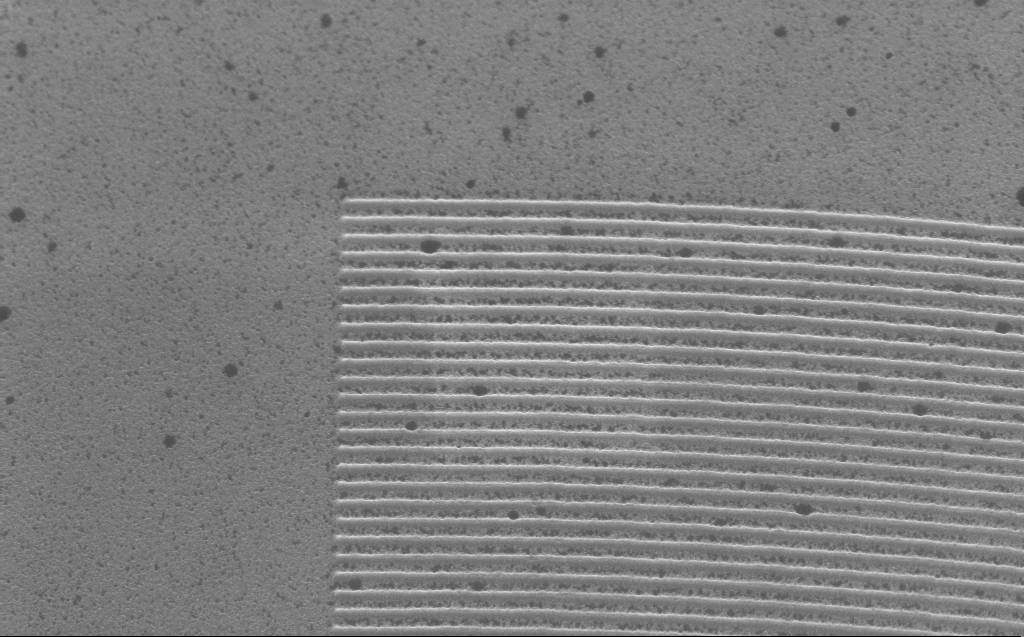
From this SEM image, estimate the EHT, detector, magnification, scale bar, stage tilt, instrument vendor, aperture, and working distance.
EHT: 5 kV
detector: InLens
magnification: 31.83 K X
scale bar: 2000 nm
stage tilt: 30°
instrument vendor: Zeiss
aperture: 30 µm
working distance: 4 mm